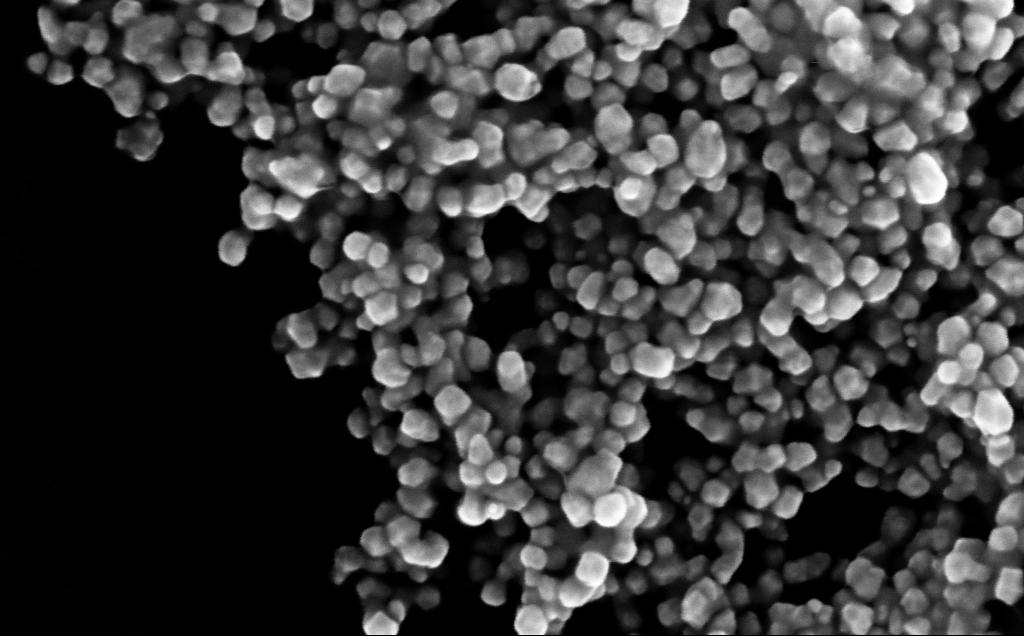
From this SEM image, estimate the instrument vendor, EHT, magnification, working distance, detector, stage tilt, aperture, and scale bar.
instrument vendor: Zeiss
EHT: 10 kV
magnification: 277.18 K X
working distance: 4 mm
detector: InLens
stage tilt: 0°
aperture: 30 µm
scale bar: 200 nm